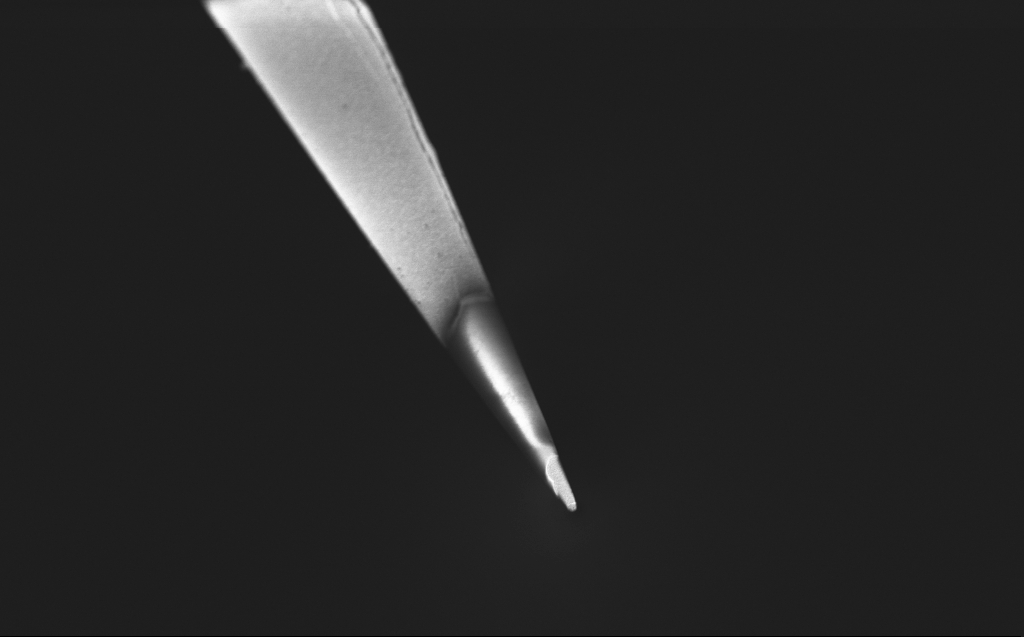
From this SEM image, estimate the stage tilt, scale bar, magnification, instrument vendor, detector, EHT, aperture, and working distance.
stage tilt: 45°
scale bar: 2000 nm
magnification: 10 K X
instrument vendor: Zeiss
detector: InLens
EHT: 0.8 kV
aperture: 30 µm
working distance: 4 mm